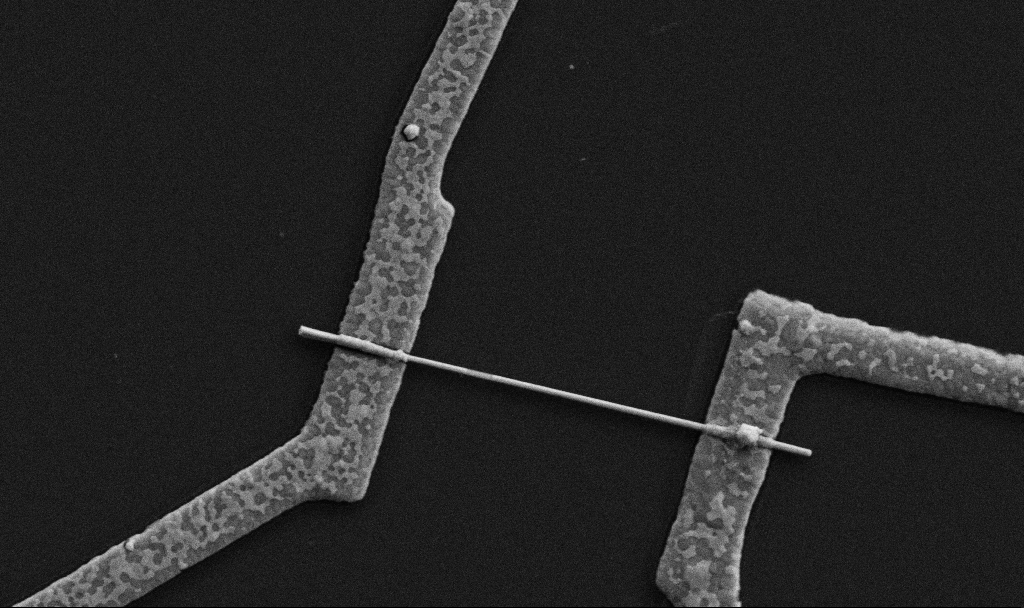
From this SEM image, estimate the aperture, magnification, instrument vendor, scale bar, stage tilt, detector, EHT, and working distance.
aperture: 30 µm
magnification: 30 K X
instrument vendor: Zeiss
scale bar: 1000 nm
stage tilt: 0°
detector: SE2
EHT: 5 kV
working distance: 8.7 mm